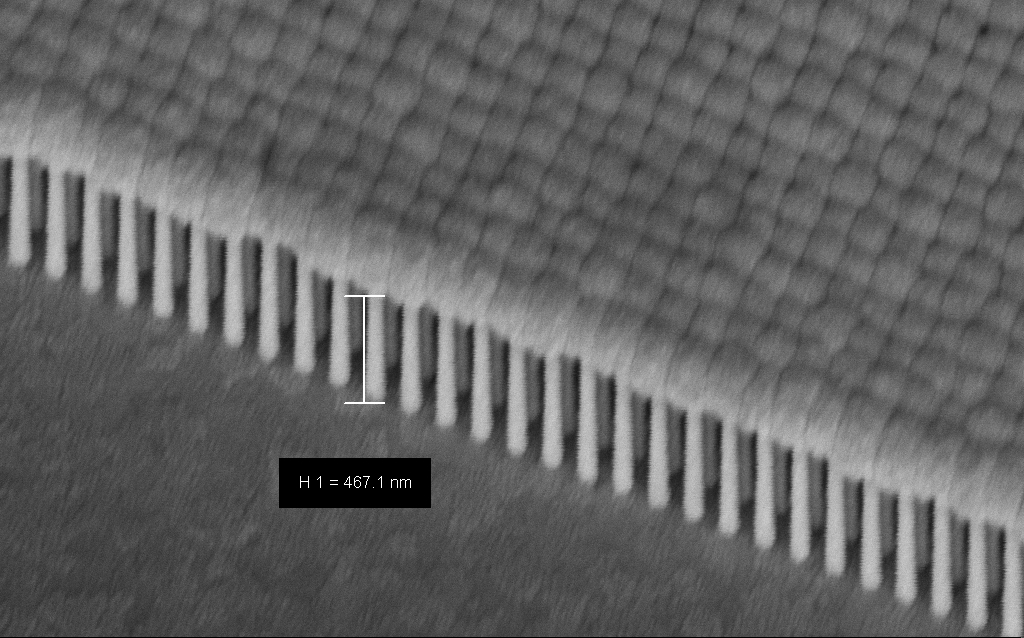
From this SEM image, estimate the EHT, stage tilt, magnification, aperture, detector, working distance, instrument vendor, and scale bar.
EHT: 1.5 kV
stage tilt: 45°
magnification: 84.11 K X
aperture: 30 µm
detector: InLens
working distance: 9.3 mm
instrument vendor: Zeiss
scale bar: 200 nm